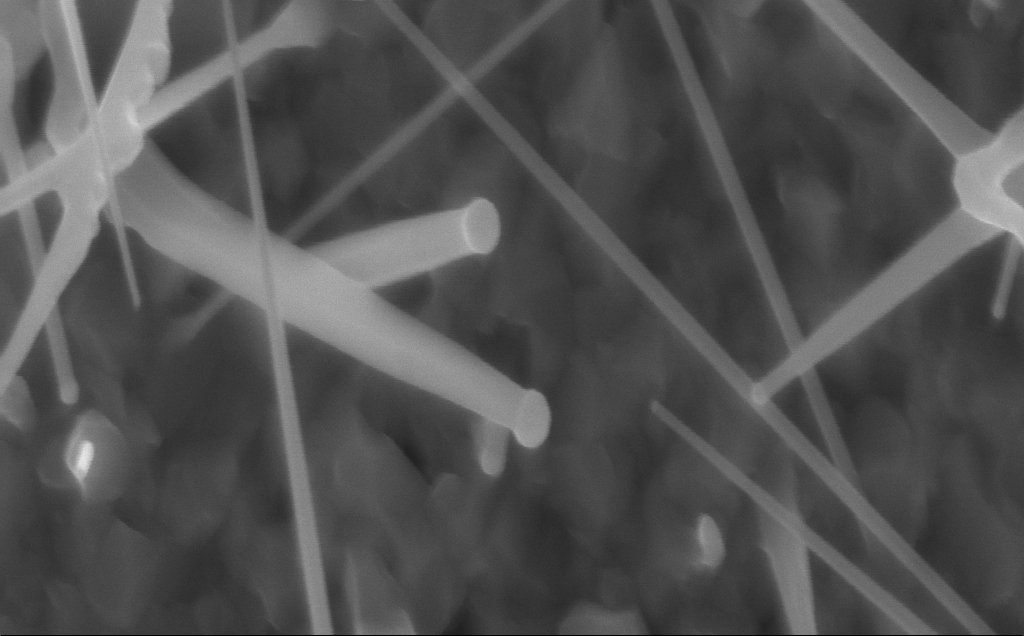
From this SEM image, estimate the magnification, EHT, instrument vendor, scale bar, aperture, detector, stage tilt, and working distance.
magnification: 106.29 K X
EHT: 10 kV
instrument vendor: Zeiss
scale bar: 200 nm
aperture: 30 µm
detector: InLens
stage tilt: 0°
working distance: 4 mm